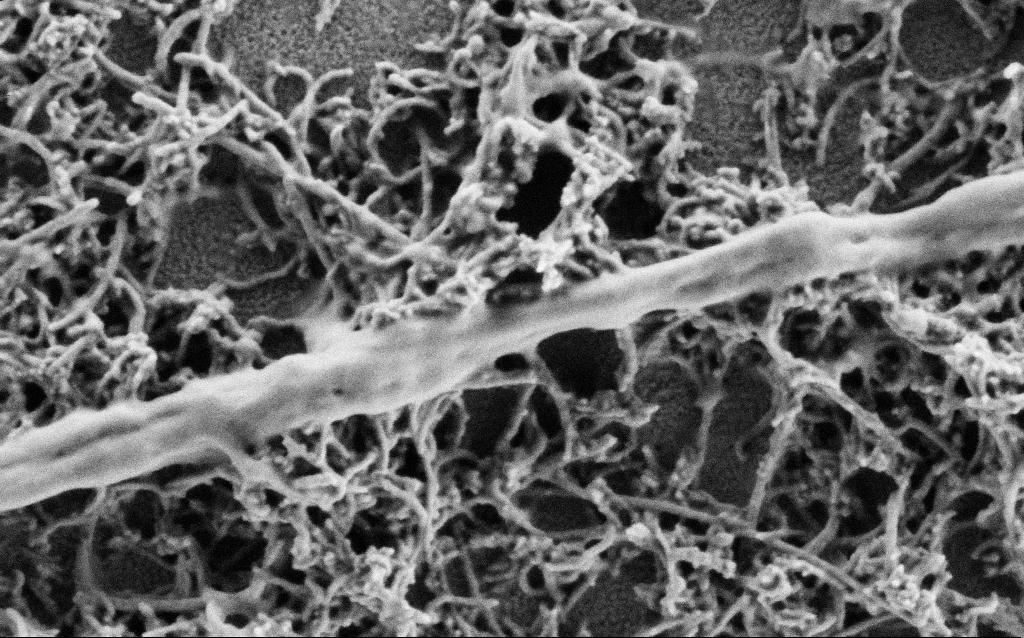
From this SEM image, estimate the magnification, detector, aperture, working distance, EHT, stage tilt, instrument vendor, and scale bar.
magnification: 100 K X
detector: SE2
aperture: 30 µm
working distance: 4 mm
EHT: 1 kV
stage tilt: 0°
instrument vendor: Zeiss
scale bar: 200 nm